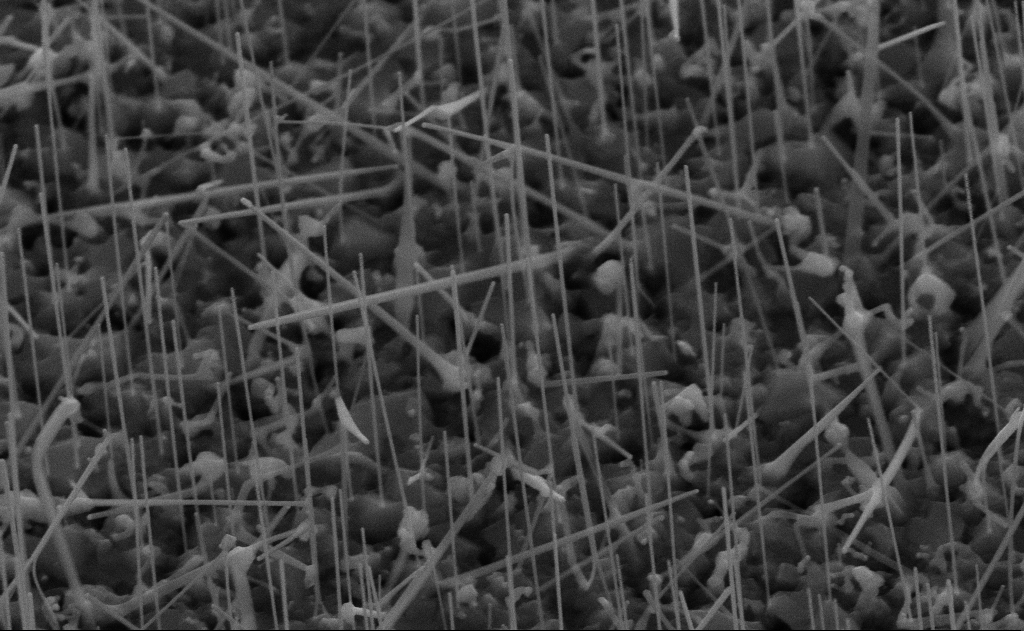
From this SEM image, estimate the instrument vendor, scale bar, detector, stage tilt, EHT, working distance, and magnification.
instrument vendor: Zeiss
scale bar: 1000 nm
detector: SE2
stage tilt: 45°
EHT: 10 kV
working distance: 11 mm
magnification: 60 K X